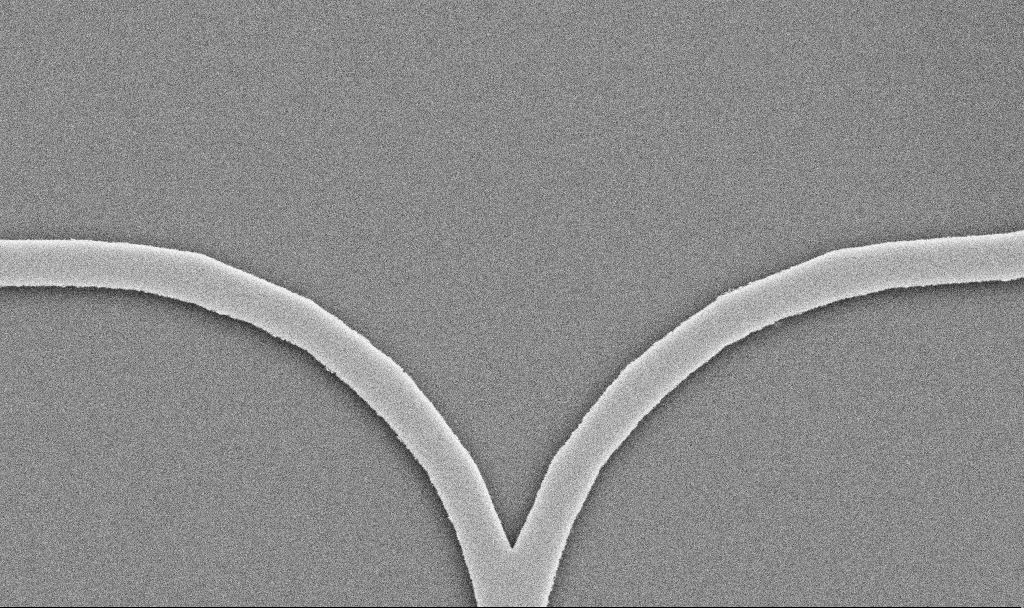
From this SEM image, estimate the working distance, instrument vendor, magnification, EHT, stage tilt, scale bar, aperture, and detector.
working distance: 10.3 mm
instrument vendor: Zeiss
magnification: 33.47 K X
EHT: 5 kV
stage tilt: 0°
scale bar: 1000 nm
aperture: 30 µm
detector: SE2